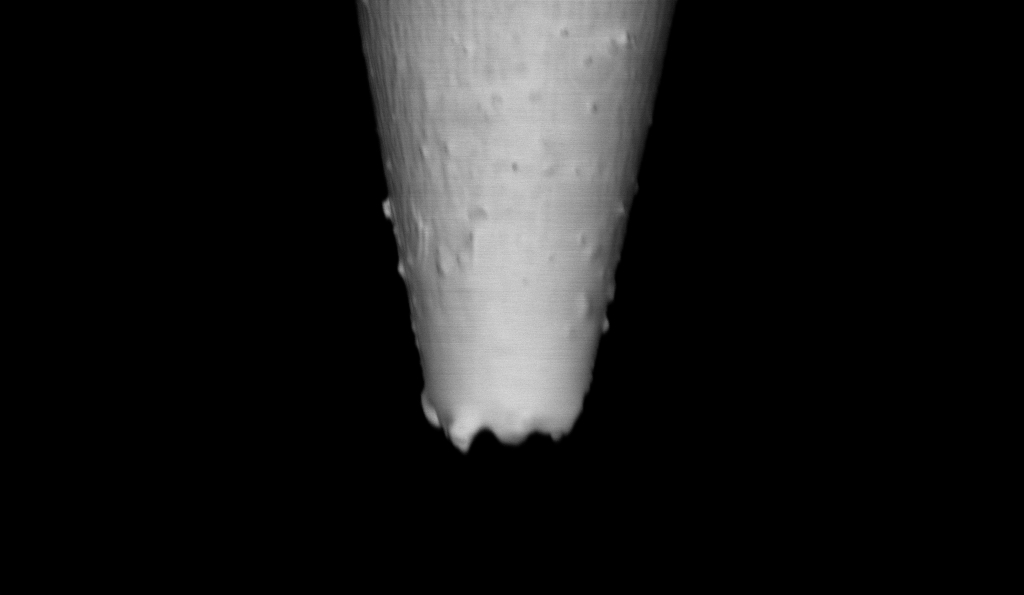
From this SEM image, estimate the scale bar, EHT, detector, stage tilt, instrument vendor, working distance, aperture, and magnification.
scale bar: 1000 nm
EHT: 1 kV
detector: InLens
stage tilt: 0°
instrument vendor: Zeiss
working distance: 6.8 mm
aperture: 30 µm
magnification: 25 K X